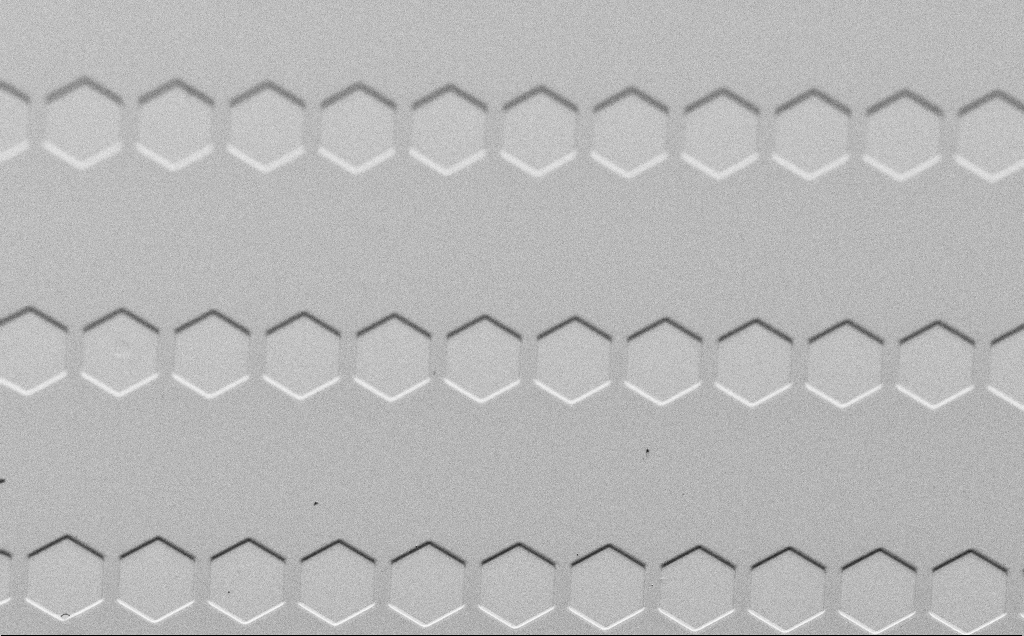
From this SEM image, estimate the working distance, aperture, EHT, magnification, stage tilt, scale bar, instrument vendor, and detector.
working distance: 4 mm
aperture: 30 µm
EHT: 1.5 kV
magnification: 0.561 K X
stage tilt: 45°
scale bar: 100000 nm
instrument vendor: Zeiss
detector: SE2